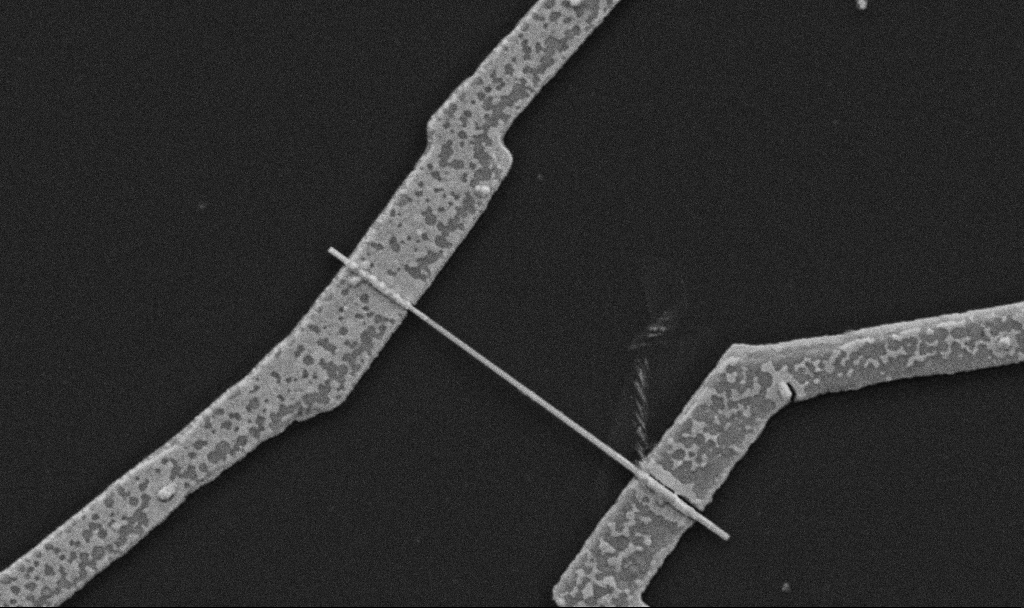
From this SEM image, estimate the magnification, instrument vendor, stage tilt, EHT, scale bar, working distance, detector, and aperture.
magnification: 30 K X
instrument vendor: Zeiss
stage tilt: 0°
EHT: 5 kV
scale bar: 1000 nm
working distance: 10.7 mm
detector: SE2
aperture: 30 µm